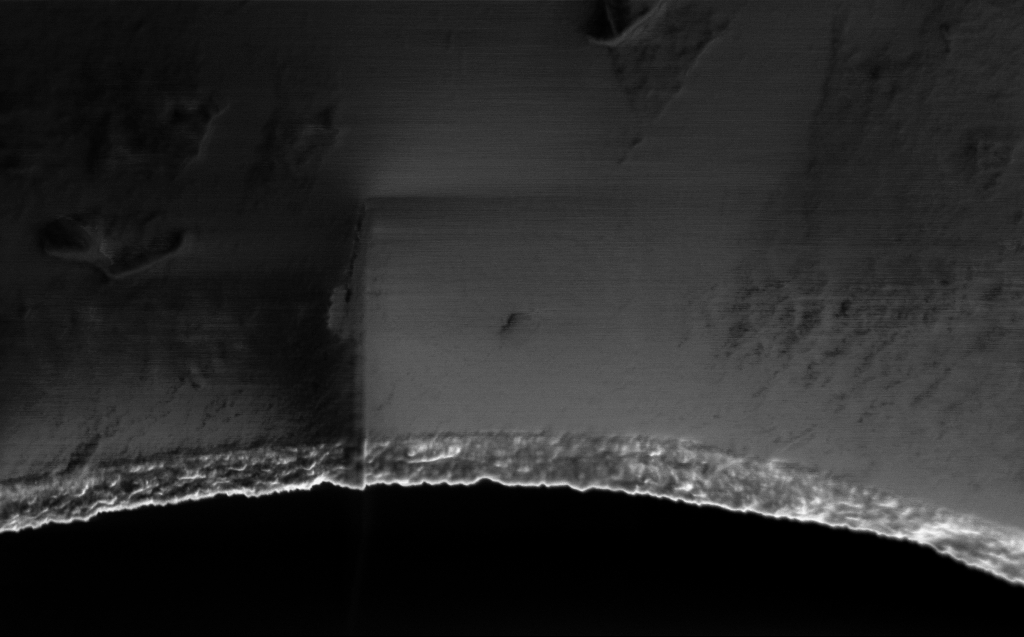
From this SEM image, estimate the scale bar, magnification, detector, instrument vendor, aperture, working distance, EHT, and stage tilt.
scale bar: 1000 nm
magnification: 40.54 K X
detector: InLens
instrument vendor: Zeiss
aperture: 30 µm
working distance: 3 mm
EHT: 1 kV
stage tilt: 45°